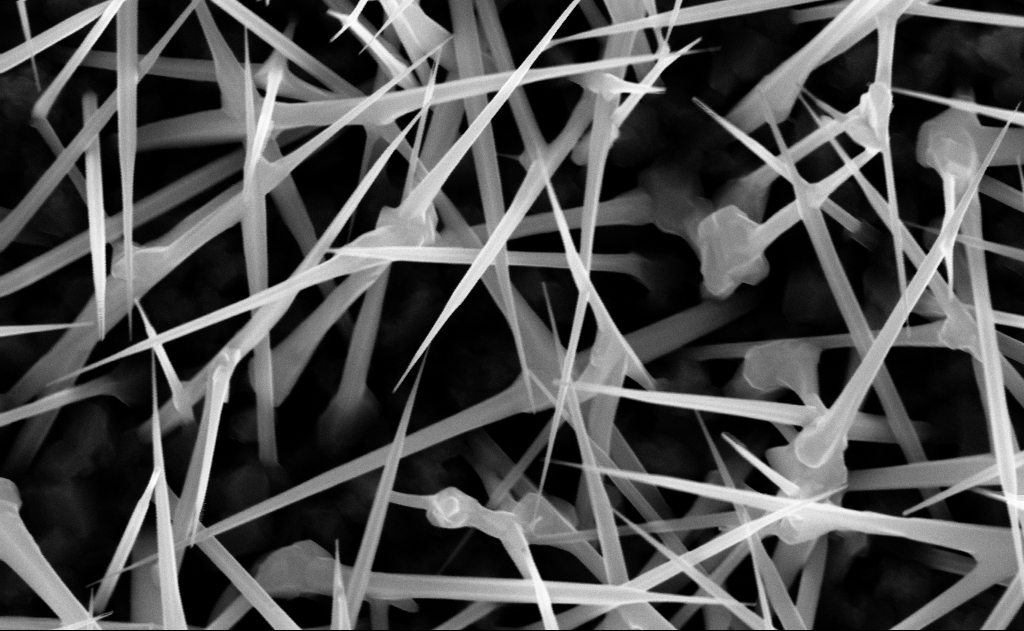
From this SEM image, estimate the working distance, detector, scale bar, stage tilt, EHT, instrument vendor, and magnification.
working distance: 9 mm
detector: InLens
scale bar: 200 nm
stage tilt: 0°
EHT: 10 kV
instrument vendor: Zeiss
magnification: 80 K X